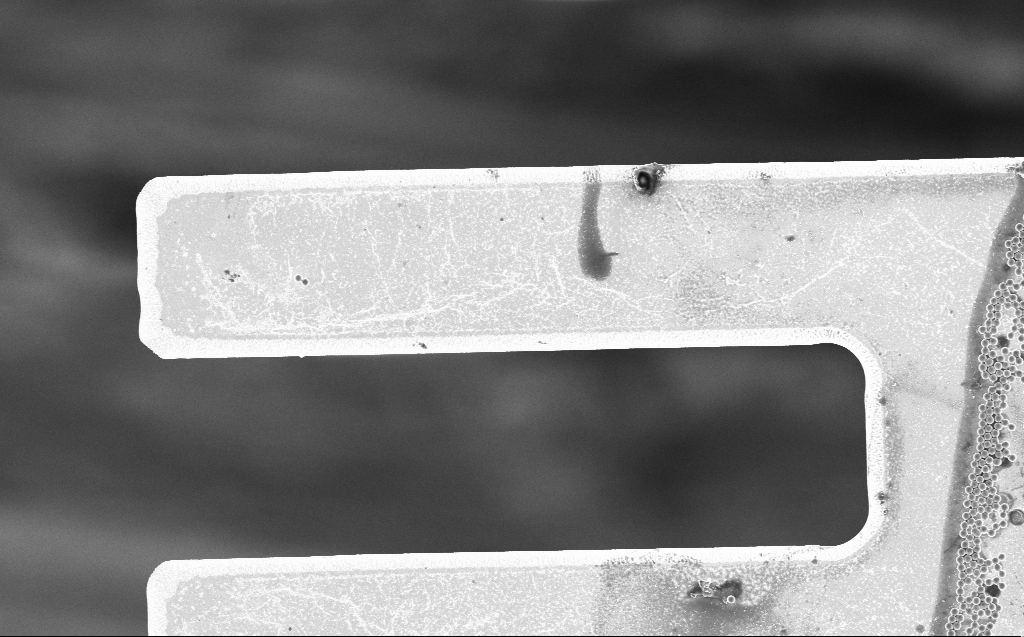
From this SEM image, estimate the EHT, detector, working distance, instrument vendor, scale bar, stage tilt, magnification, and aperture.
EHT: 3 kV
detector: InLens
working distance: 7 mm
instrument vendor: Zeiss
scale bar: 20000 nm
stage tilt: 0°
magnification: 3.54 K X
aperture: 30 µm